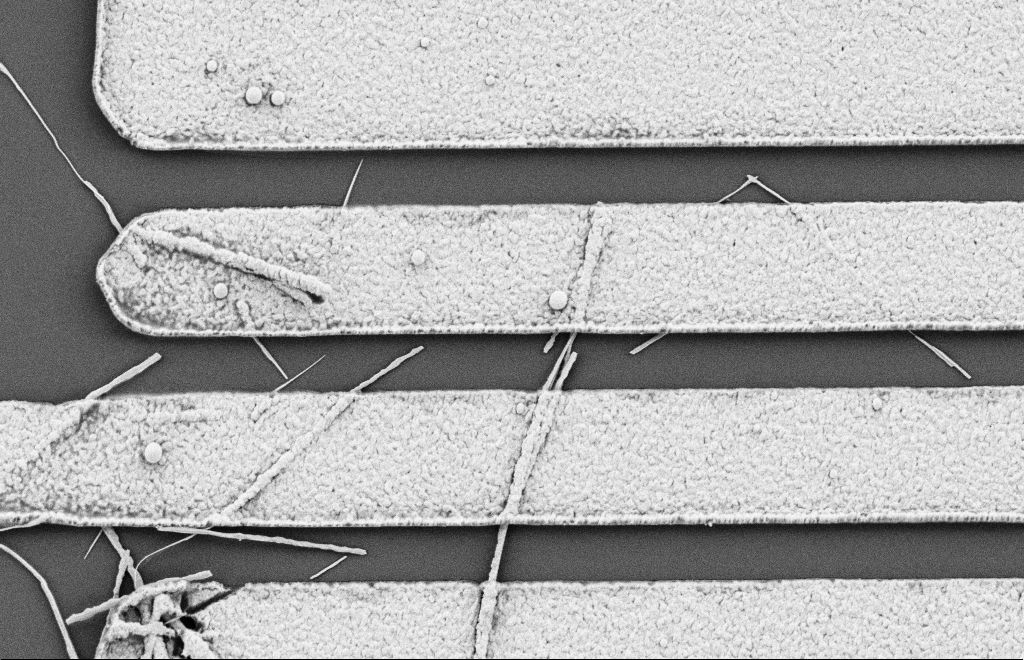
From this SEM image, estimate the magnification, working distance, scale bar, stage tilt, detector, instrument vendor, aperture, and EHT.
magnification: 17.23 K X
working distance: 10 mm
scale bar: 2000 nm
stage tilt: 0°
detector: SE2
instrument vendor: Zeiss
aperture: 20 µm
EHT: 2 kV